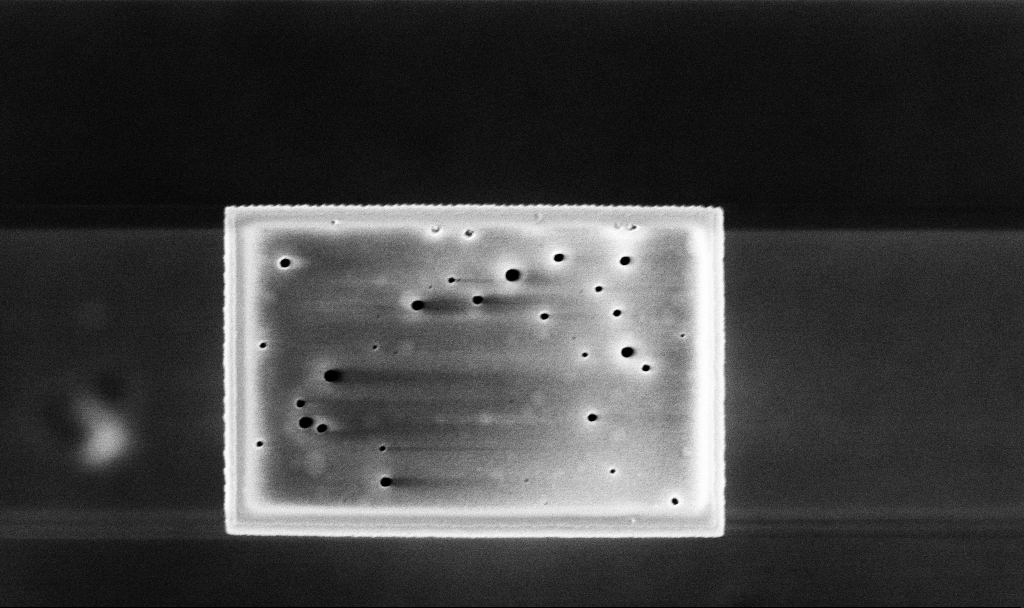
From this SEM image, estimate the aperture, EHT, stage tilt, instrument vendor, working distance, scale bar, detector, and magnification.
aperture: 30 µm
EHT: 5 kV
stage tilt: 0°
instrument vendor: Zeiss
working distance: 3.3 mm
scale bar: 1000 nm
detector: InLens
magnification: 42.02 K X